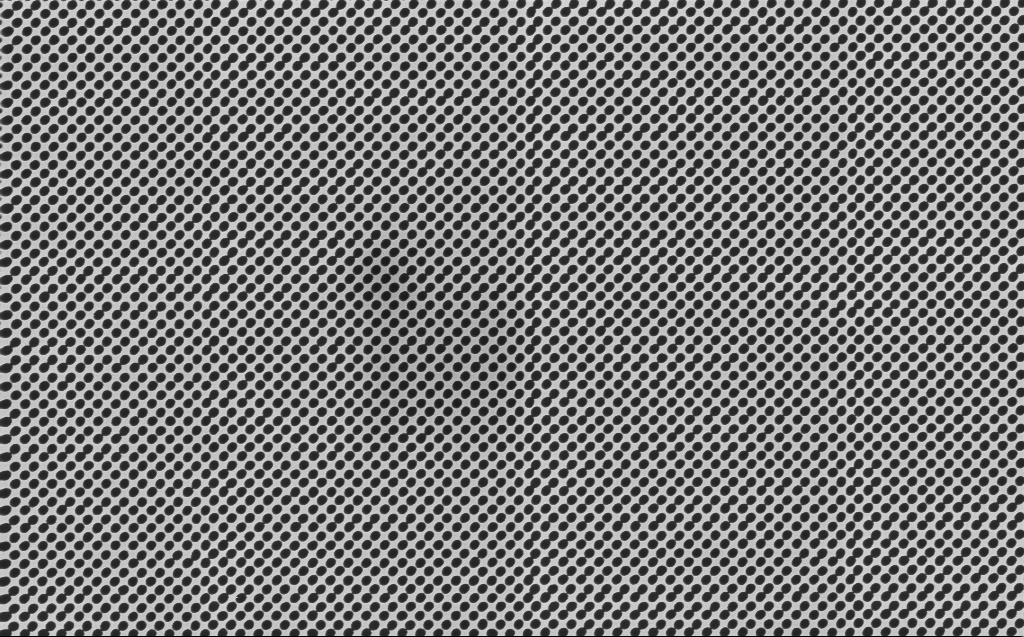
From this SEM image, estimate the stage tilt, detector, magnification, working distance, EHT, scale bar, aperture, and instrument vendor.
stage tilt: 0°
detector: InLens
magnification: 26.24 K X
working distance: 7 mm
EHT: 5 kV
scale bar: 1000 nm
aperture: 30 µm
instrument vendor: Zeiss